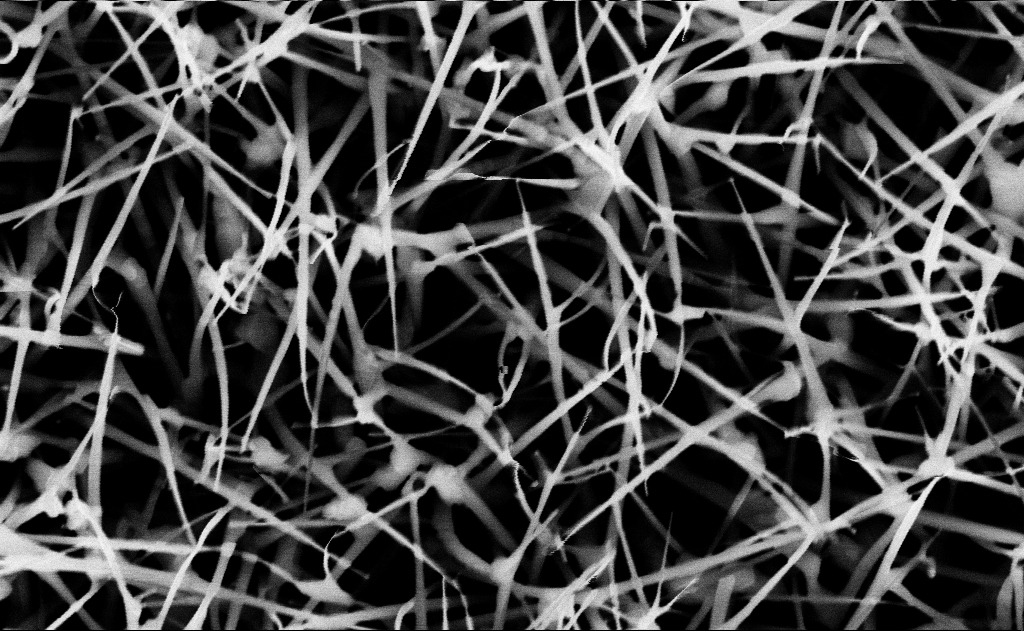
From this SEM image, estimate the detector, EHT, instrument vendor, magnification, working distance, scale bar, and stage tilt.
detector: InLens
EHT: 10 kV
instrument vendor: Zeiss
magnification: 60 K X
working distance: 13 mm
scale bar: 1000 nm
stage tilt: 0°